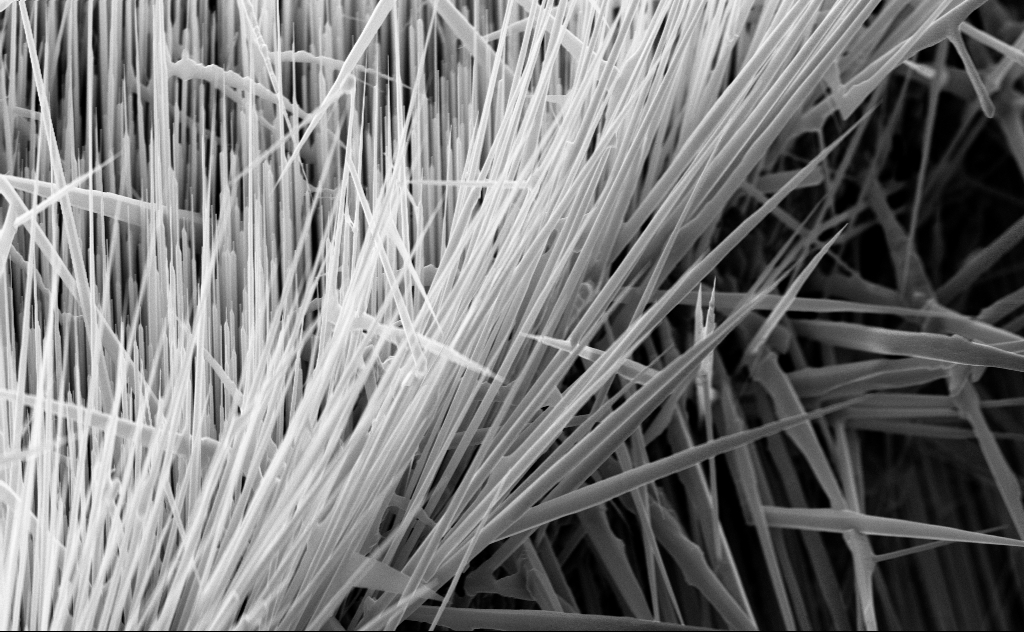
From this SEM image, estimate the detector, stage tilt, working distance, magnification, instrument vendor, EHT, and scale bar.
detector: InLens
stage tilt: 45°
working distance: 6 mm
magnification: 15.99 K X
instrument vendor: Zeiss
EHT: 10 kV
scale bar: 2000 nm